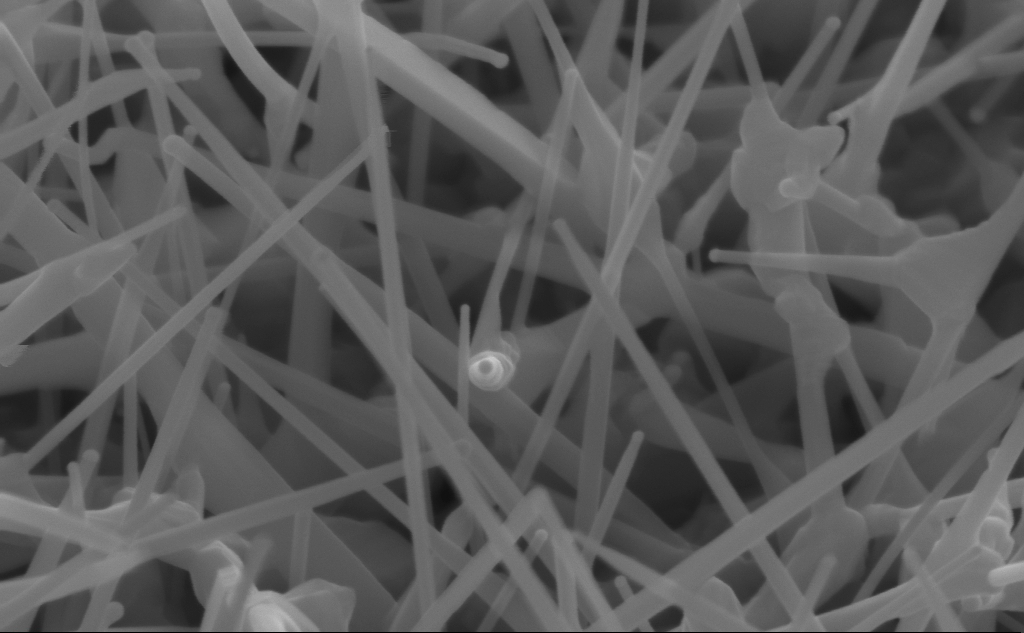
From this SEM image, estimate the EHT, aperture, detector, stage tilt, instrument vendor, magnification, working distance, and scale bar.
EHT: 10 kV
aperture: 30 µm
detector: InLens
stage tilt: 45°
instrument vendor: Zeiss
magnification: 150 K X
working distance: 4 mm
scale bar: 200 nm